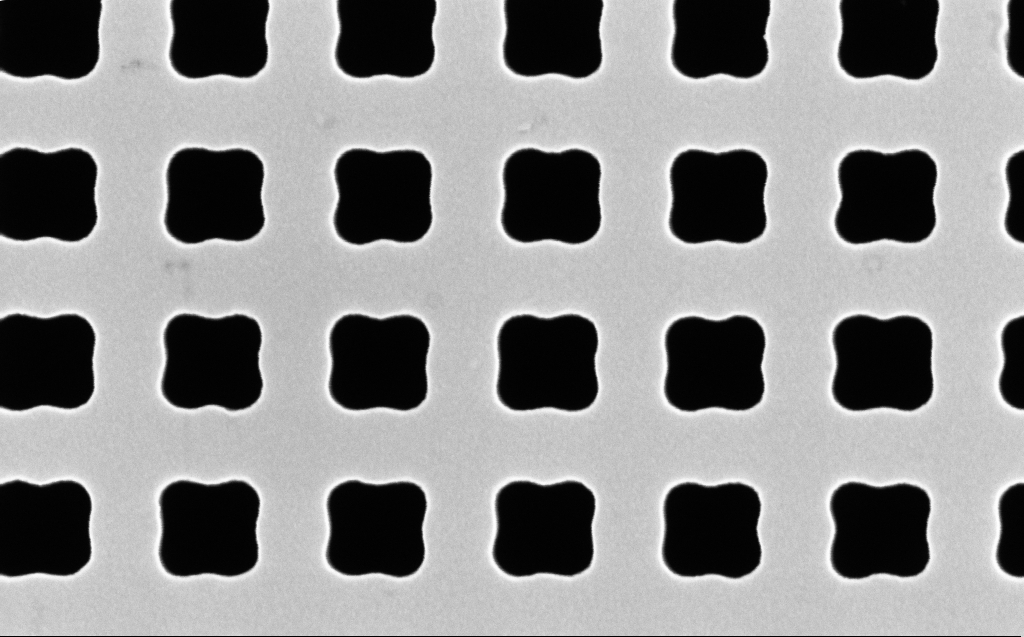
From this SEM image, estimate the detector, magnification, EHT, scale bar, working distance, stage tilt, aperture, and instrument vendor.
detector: InLens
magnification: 124.06 K X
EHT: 10 kV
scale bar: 200 nm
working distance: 7 mm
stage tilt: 0°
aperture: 30 µm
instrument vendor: Zeiss